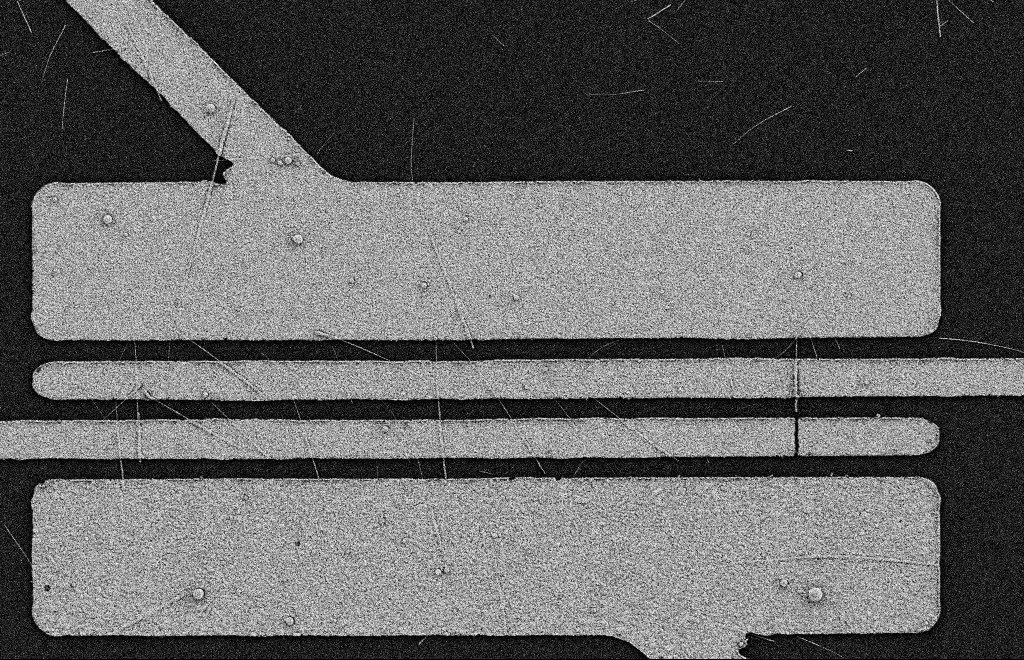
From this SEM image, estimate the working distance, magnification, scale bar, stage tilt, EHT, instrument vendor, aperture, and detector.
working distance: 11 mm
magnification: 5.41 K X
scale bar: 2000 nm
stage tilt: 0°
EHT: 2 kV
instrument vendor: Zeiss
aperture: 20 µm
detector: SE2